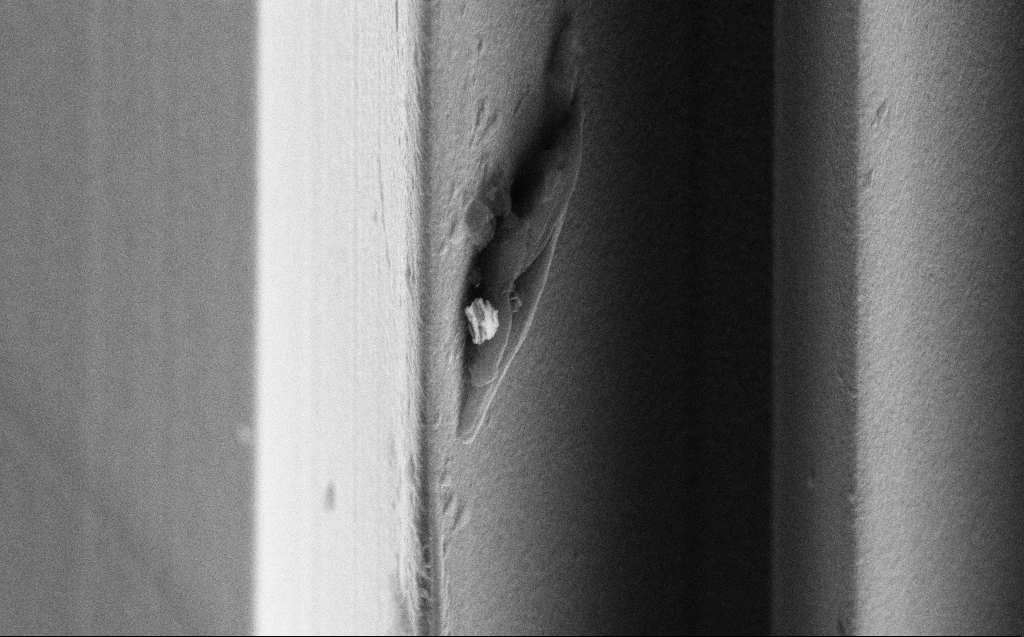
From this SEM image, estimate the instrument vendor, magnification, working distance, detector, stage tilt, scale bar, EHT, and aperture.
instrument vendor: Zeiss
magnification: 14.11 K X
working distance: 9 mm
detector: SE2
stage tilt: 45°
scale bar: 1000 nm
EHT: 10 kV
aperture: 30 µm